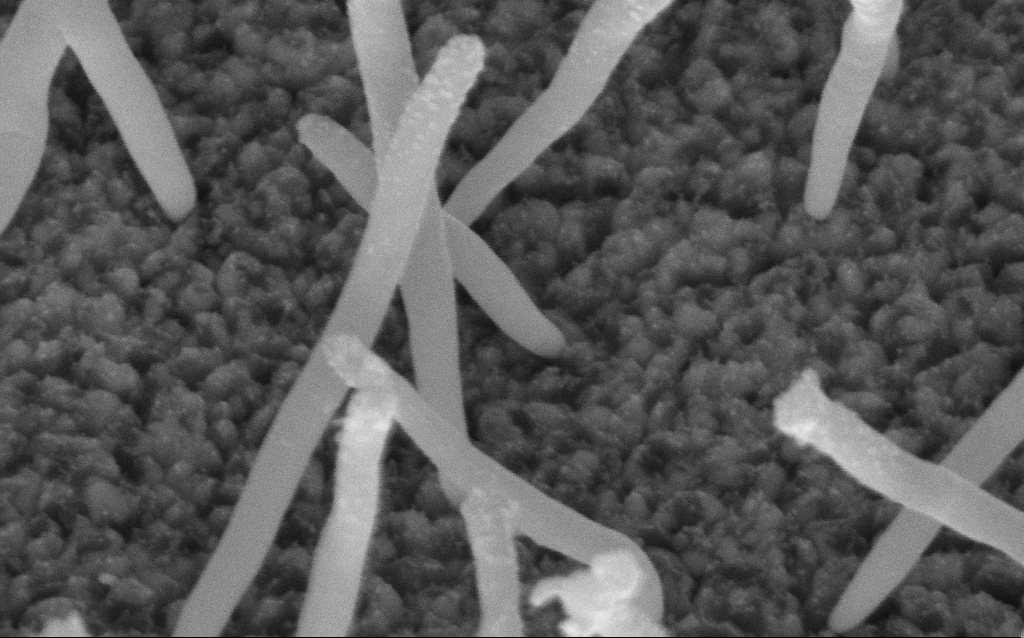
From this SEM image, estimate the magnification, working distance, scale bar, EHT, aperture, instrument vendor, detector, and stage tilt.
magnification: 200 K X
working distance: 8.3 mm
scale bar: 100 nm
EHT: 5 kV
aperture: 30 µm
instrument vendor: Zeiss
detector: InLens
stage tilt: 45°